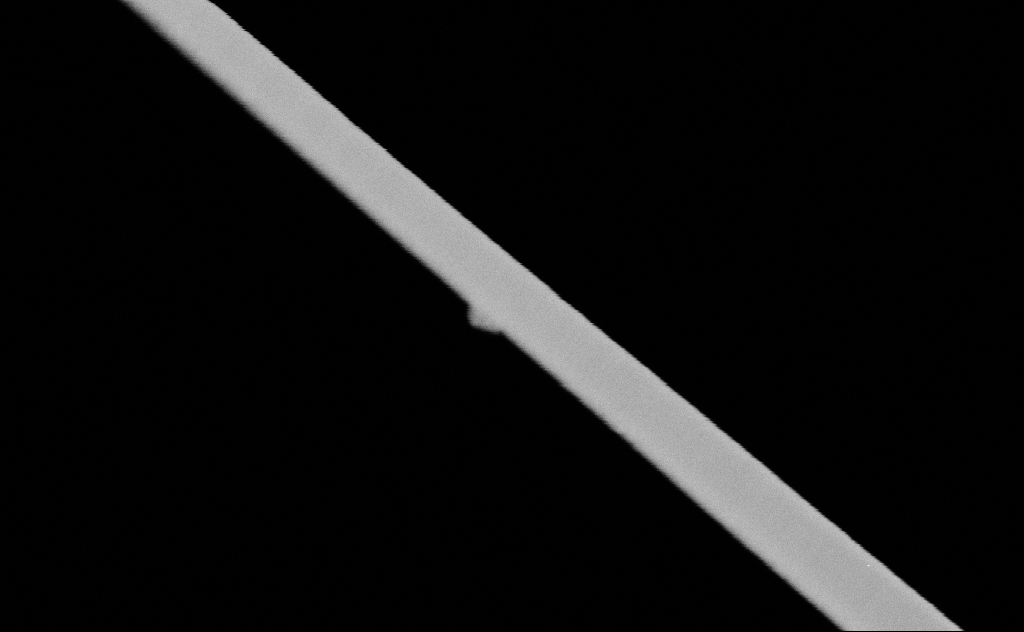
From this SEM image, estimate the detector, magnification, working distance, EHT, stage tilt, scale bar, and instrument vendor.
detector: SE2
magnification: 316.08 K X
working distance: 10 mm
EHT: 20 kV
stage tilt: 0°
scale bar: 200 nm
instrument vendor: Zeiss